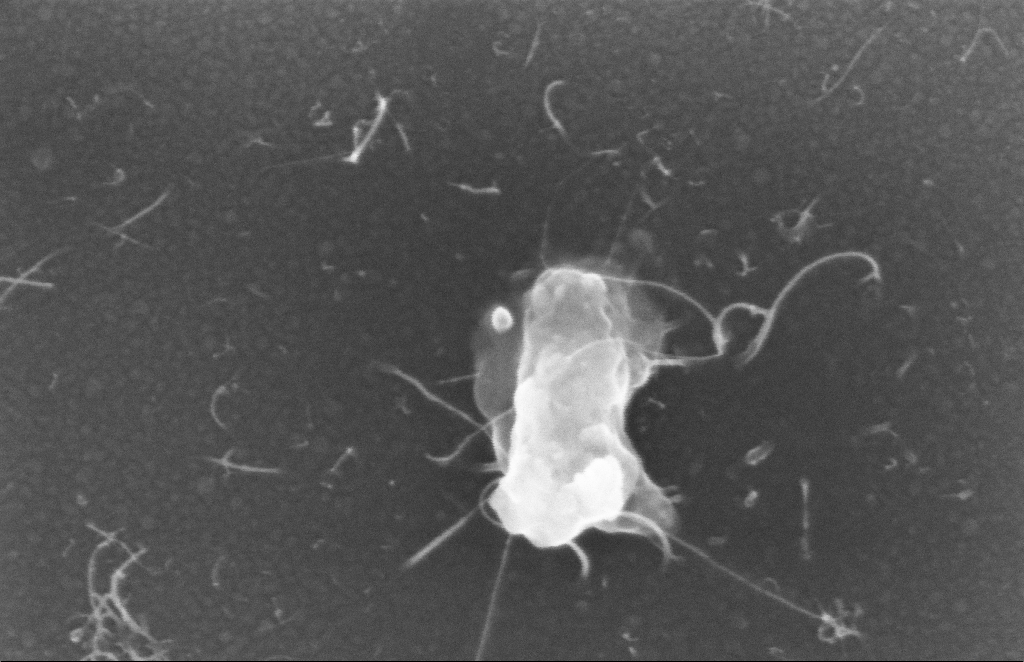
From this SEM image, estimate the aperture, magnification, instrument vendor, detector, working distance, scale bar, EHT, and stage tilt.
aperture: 20 µm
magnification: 264.51 K X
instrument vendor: Zeiss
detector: InLens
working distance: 8 mm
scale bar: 100 nm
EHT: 5 kV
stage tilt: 0°